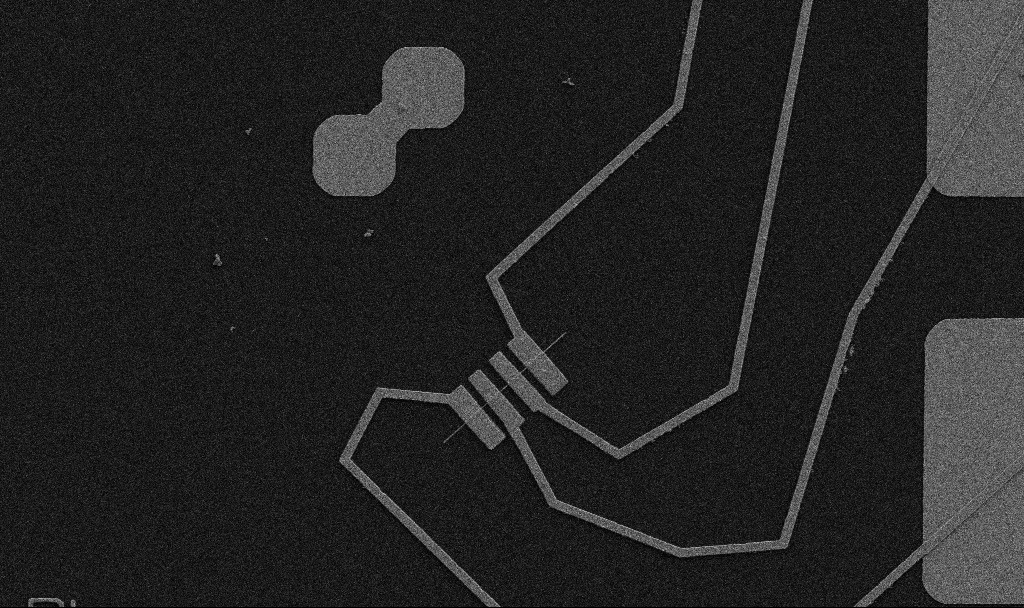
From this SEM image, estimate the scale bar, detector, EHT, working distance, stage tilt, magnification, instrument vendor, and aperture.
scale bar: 10000 nm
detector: SE2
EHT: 5 kV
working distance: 10.7 mm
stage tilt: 0°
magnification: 5 K X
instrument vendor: Zeiss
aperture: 30 µm